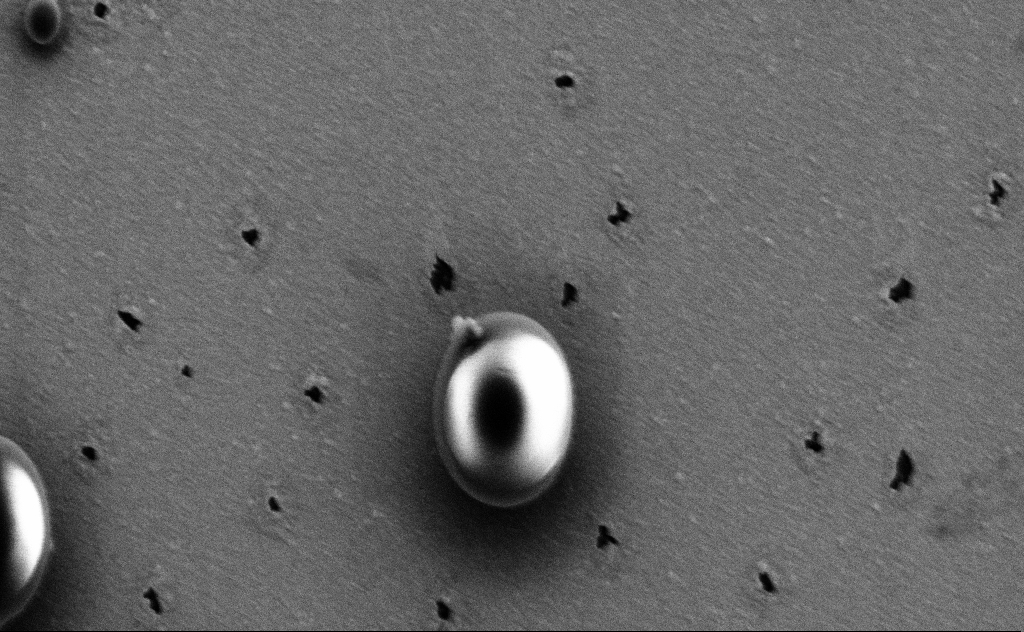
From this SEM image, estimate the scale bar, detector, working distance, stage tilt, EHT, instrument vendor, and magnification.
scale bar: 2000 nm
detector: SE2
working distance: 10 mm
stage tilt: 0°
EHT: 3 kV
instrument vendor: Zeiss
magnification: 26.01 K X